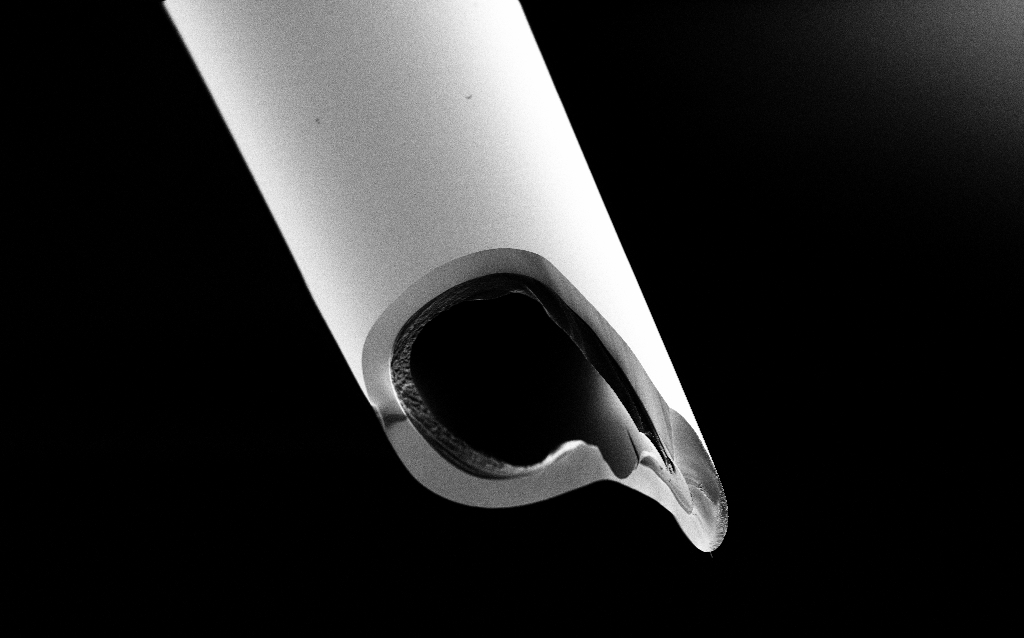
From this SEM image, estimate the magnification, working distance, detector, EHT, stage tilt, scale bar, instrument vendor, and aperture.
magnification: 2.5 K X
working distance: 6.4 mm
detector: SE2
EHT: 1 kV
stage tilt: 45°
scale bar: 10000 nm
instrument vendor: Zeiss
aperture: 30 µm